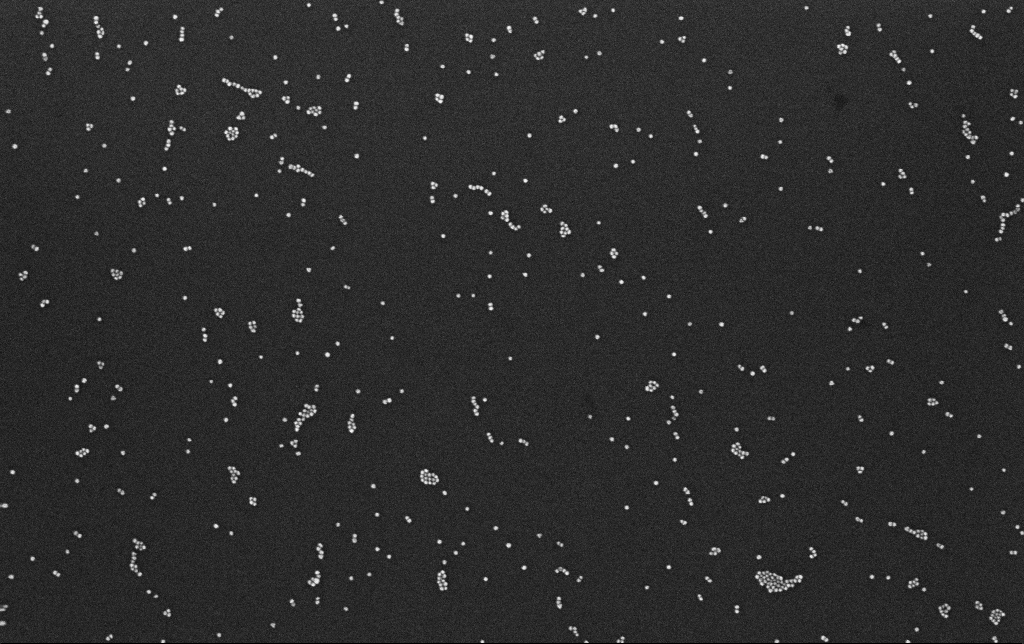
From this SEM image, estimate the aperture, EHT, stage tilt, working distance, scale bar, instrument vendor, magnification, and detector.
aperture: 30 µm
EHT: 10 kV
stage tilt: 0°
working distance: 3.1 mm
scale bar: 200 nm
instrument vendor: Zeiss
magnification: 100 K X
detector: InLens